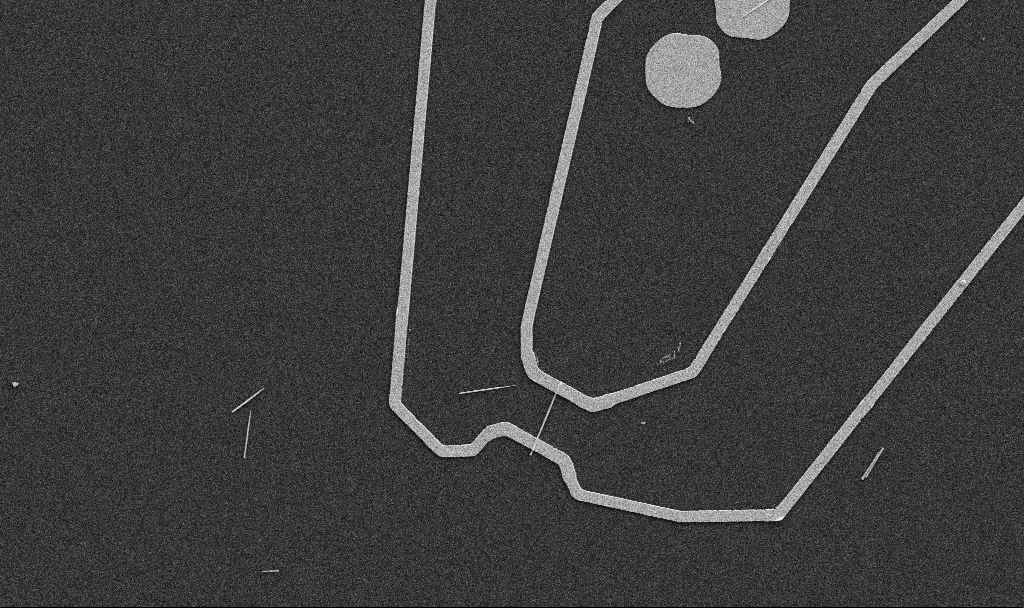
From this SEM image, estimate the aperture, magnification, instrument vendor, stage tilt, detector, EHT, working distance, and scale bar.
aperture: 30 µm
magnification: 5 K X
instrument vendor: Zeiss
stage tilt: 0°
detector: SE2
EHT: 5 kV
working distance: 10.7 mm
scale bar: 10000 nm